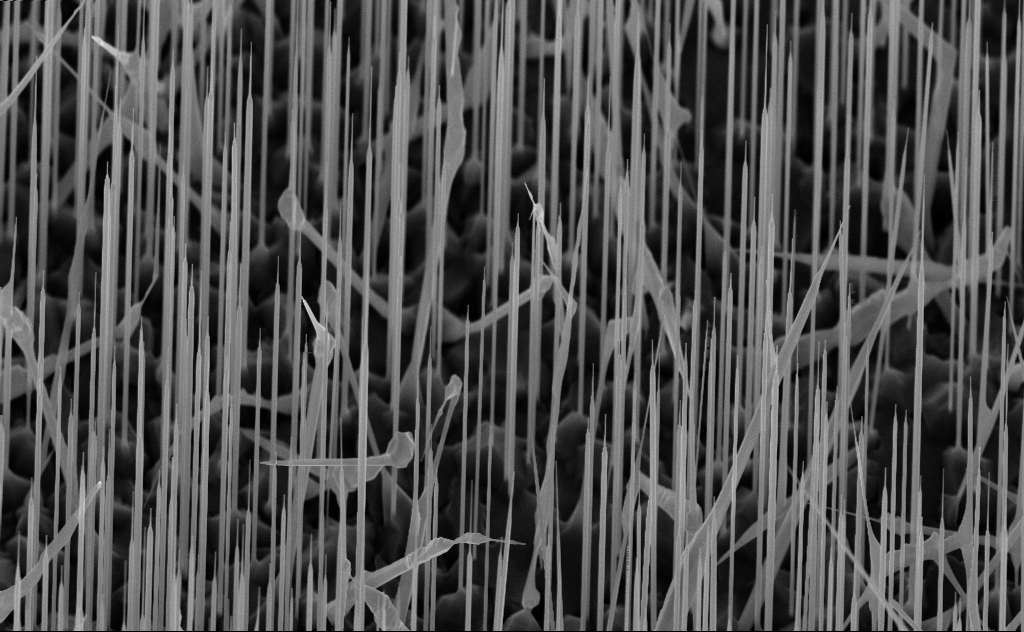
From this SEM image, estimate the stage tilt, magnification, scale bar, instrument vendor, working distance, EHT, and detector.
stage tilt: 44.9°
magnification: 20 K X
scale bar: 2000 nm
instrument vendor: Zeiss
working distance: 7 mm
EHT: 10 kV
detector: InLens